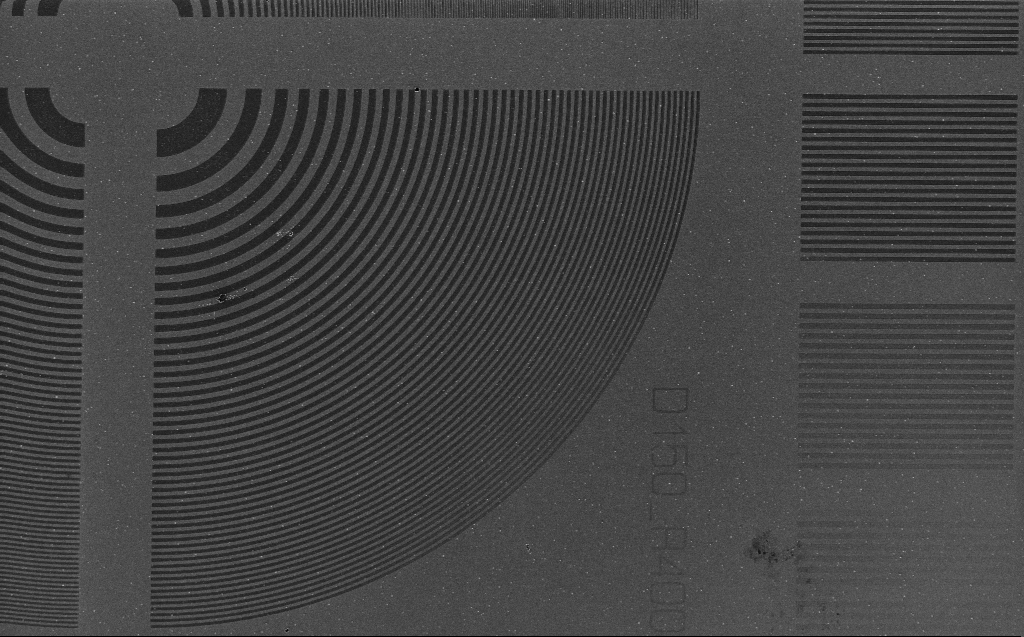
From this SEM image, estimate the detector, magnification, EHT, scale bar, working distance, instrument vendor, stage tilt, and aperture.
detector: InLens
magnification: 2.64 K X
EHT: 1.2 kV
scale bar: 10000 nm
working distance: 5 mm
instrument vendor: Zeiss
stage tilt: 0°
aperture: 30 µm